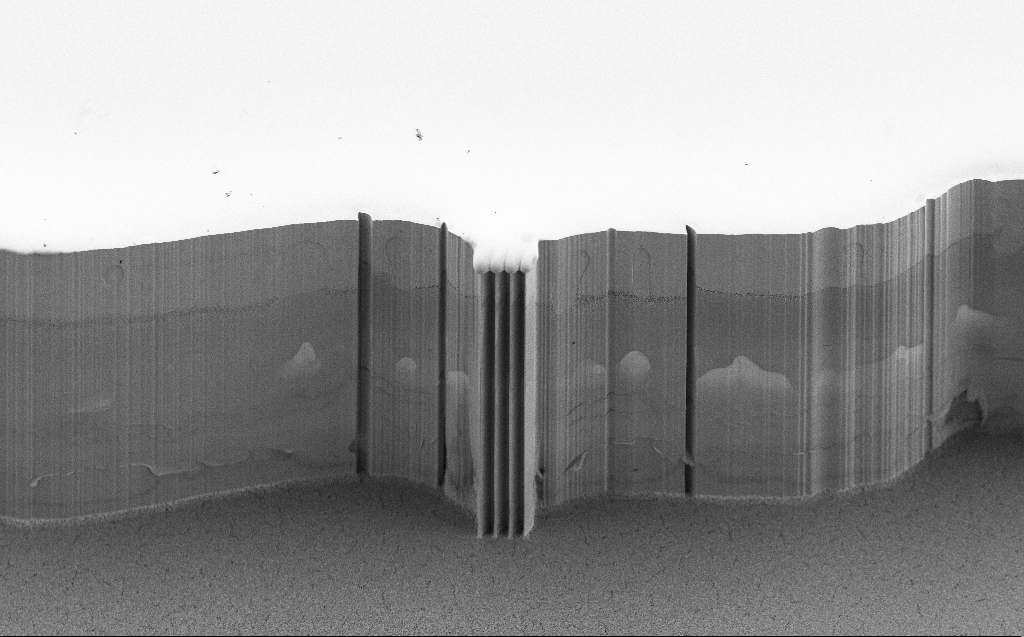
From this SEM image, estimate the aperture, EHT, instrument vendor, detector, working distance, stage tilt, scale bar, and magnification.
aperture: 30 µm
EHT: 5 kV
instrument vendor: Zeiss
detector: SE2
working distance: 5 mm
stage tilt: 45°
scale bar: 100000 nm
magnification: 0.477 K X